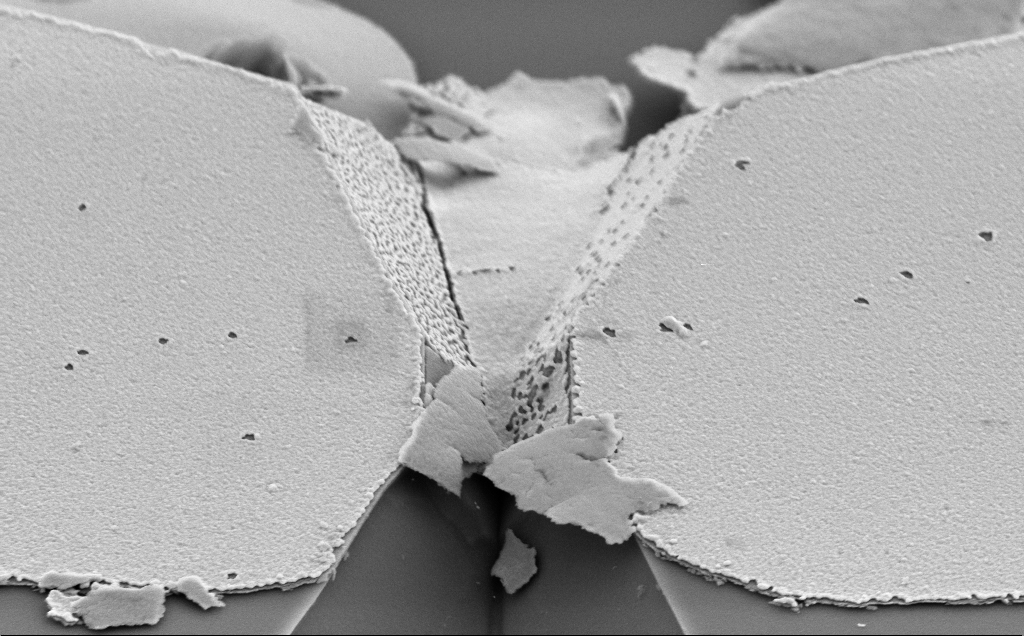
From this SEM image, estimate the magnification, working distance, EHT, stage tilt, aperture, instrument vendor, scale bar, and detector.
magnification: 24.83 K X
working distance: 10 mm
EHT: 5 kV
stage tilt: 50°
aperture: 30 µm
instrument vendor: Zeiss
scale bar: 1000 nm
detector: SE2